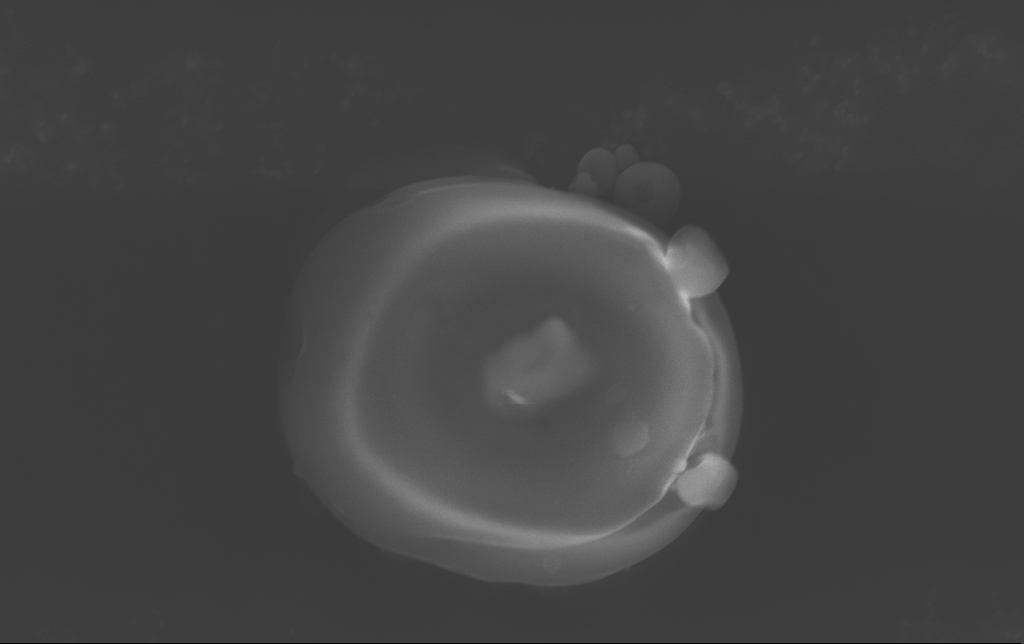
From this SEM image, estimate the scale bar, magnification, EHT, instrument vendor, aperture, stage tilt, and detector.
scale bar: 2000 nm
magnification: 20.95 K X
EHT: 15 kV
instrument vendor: Zeiss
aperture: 30 µm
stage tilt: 0°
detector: InLens